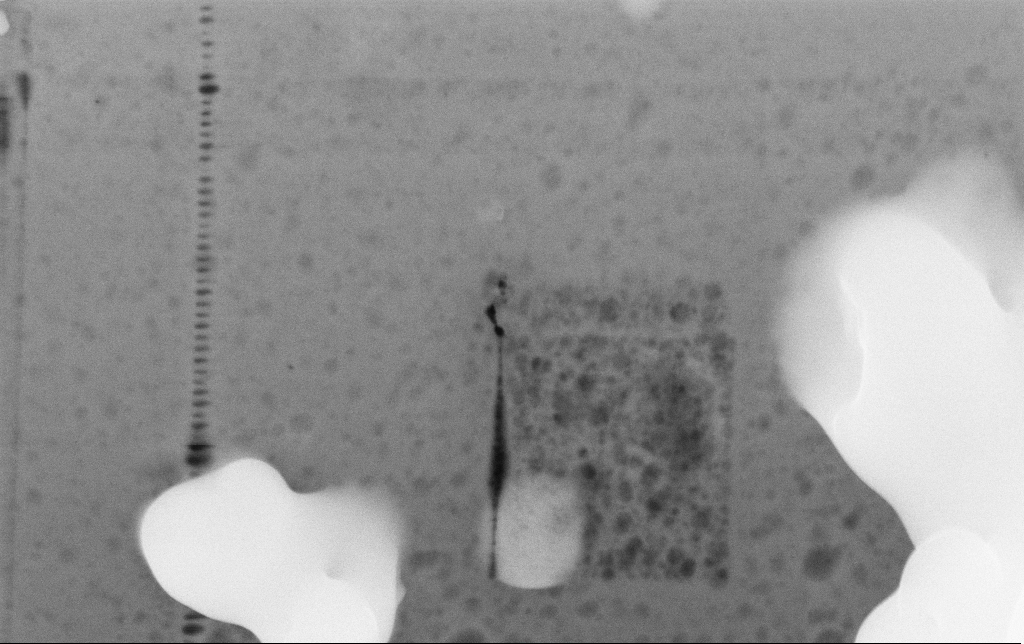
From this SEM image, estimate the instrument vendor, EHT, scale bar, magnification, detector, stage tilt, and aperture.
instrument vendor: Zeiss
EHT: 15 kV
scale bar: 1000 nm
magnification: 60.09 K X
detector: InLens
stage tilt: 0°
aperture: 30 µm